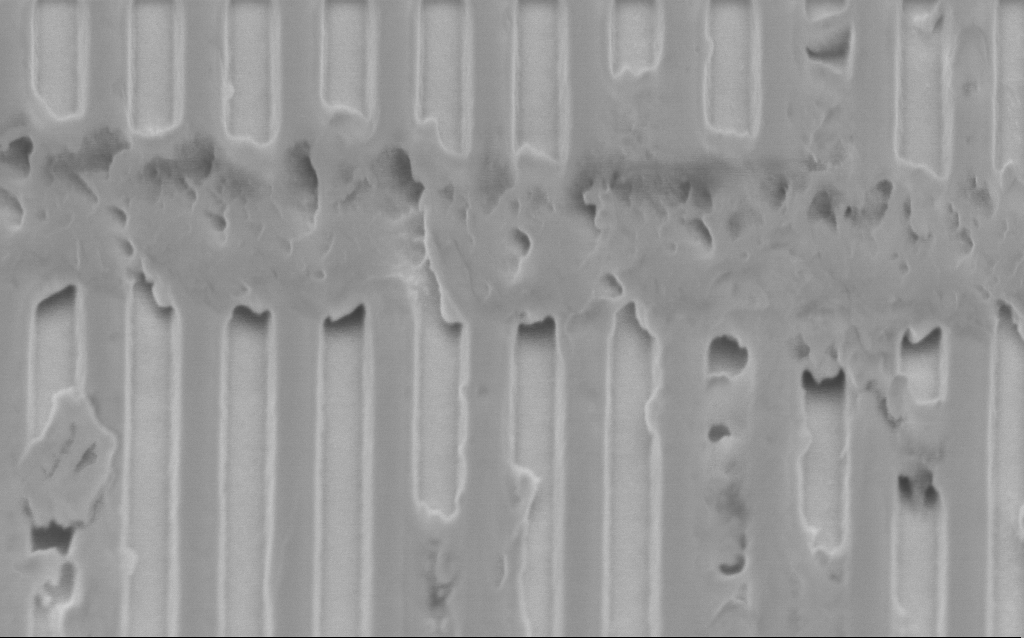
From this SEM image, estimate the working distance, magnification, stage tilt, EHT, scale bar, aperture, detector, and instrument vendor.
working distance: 2.7 mm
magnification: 71.12 K X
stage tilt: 45°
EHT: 2 kV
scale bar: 1000 nm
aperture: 30 µm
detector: InLens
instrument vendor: Zeiss